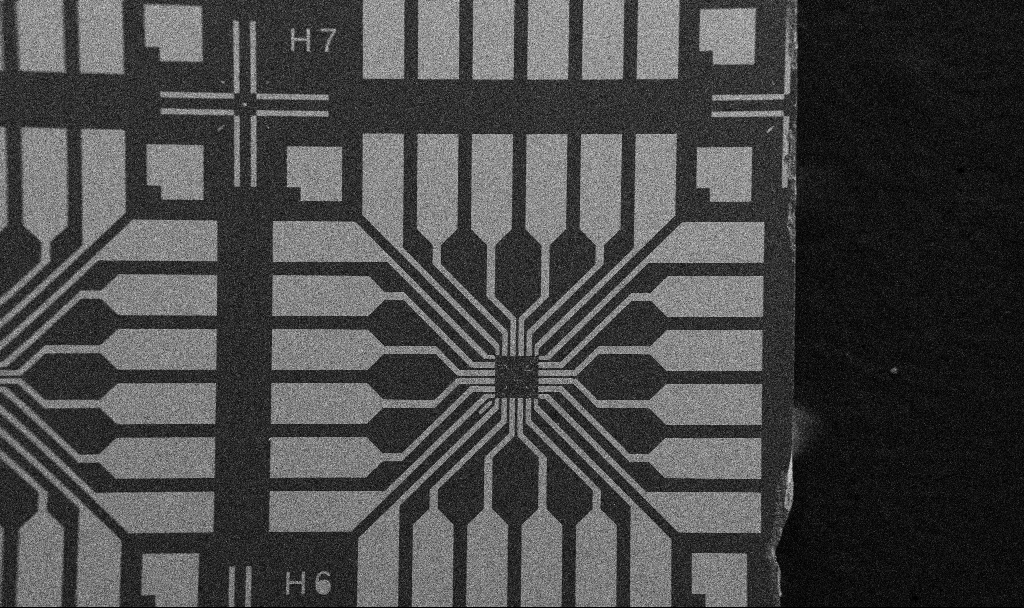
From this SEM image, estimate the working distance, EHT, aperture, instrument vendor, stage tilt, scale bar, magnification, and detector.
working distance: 10.7 mm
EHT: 5 kV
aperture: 30 µm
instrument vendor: Zeiss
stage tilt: -0°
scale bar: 200000 nm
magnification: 0.1 K X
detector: SE2